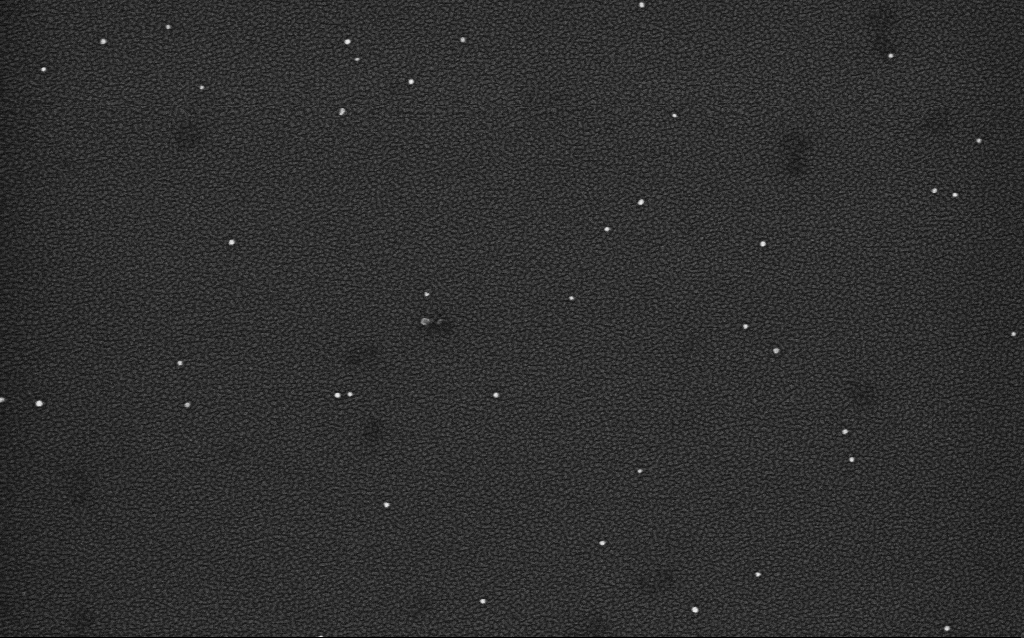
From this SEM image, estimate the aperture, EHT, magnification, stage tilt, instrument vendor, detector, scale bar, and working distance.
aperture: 30 µm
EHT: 4 kV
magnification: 100 K X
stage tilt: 0°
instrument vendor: Zeiss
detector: InLens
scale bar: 200 nm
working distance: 2.1 mm